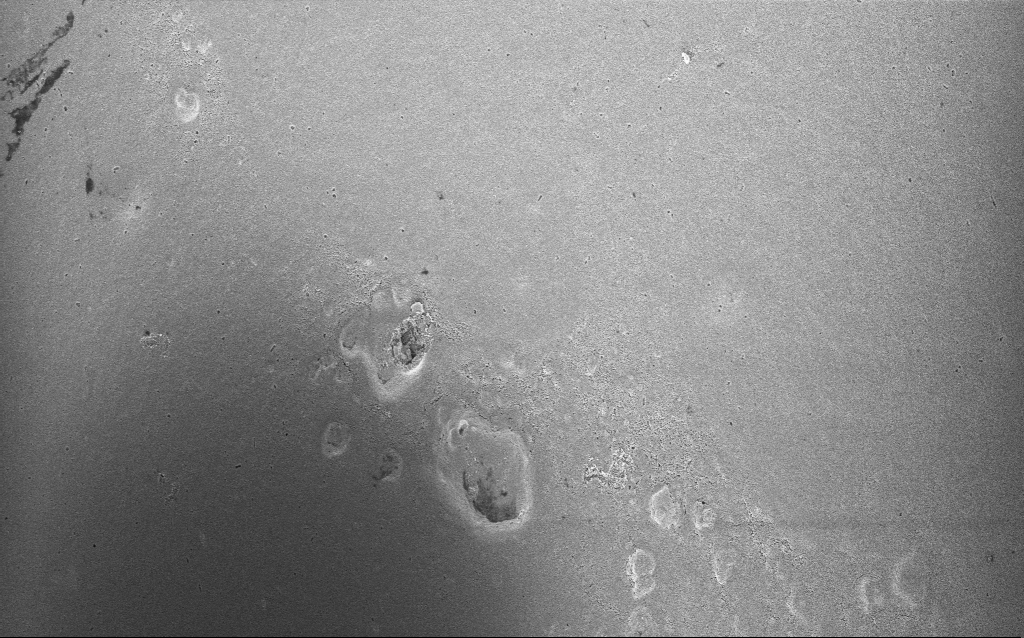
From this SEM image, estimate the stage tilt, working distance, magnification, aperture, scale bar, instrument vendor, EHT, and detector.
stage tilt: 0°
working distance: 2.7 mm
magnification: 1.69 K X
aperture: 30 µm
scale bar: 10000 nm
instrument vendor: Zeiss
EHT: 3 kV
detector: InLens